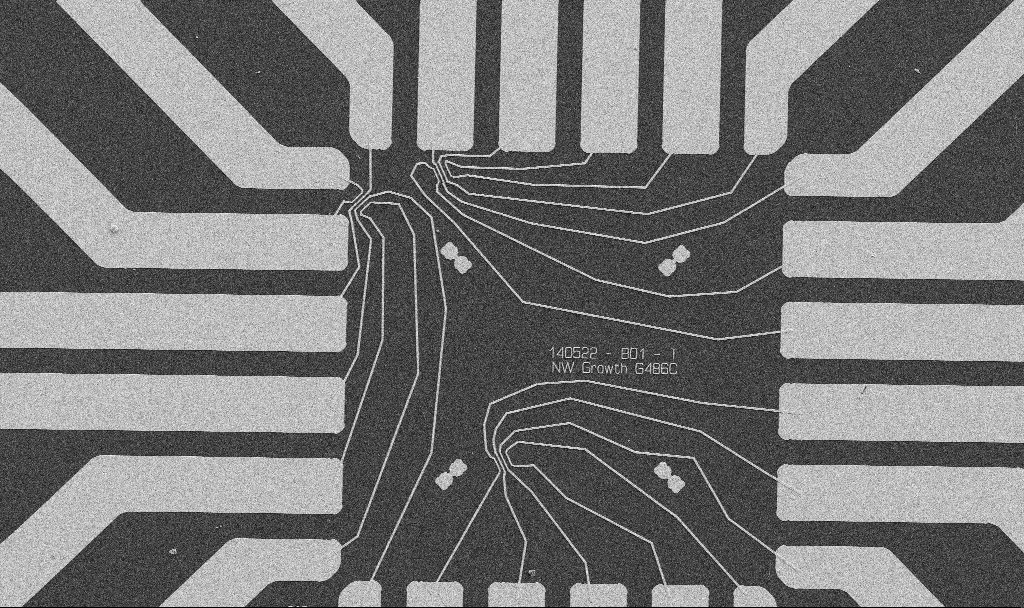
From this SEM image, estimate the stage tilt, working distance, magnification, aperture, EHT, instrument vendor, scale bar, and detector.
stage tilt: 0°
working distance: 10.7 mm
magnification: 1 K X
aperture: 30 µm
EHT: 5 kV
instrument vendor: Zeiss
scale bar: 20000 nm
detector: SE2